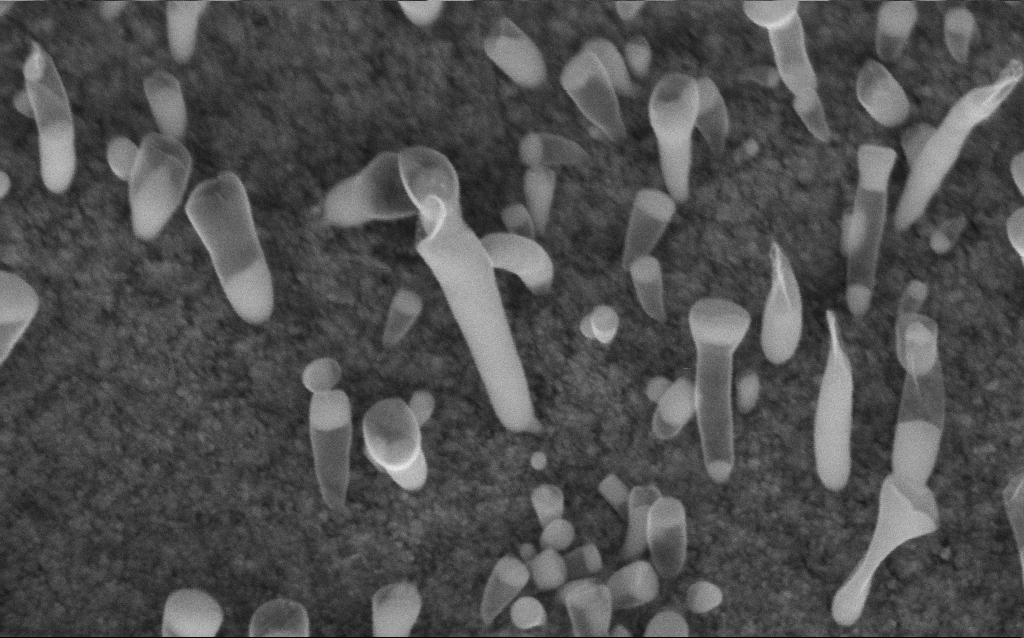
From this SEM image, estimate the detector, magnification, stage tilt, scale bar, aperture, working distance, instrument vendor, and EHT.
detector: InLens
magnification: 169.12 K X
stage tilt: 45°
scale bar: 200 nm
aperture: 30 µm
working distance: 6.6 mm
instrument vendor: Zeiss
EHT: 5 kV